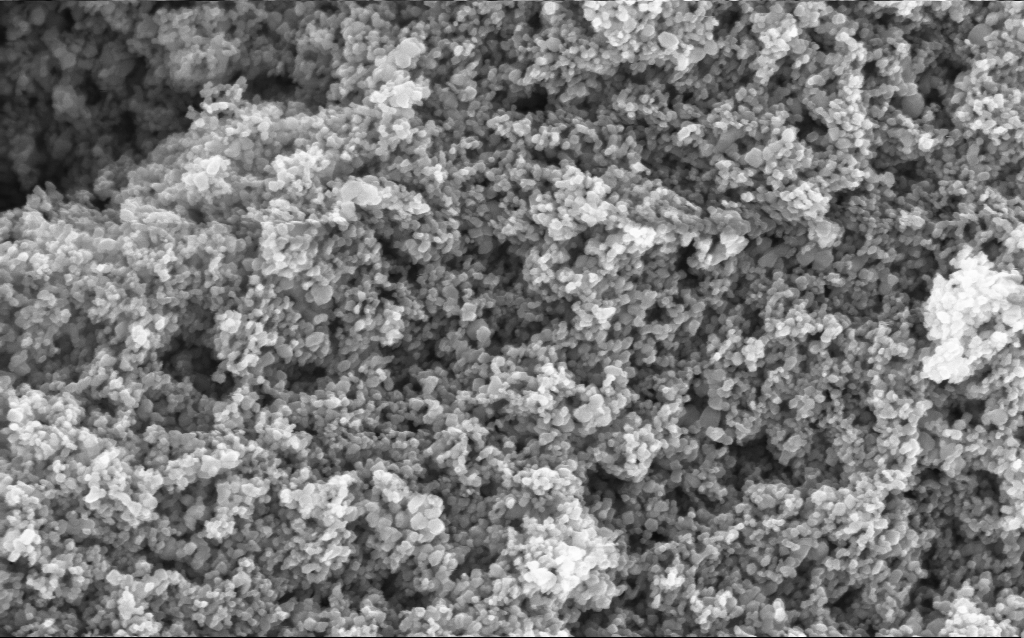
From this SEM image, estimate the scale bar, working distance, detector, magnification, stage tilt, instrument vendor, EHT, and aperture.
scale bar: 200 nm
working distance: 4.5 mm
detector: InLens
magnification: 114.64 K X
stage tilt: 0°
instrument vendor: Zeiss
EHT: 5 kV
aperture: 30 µm